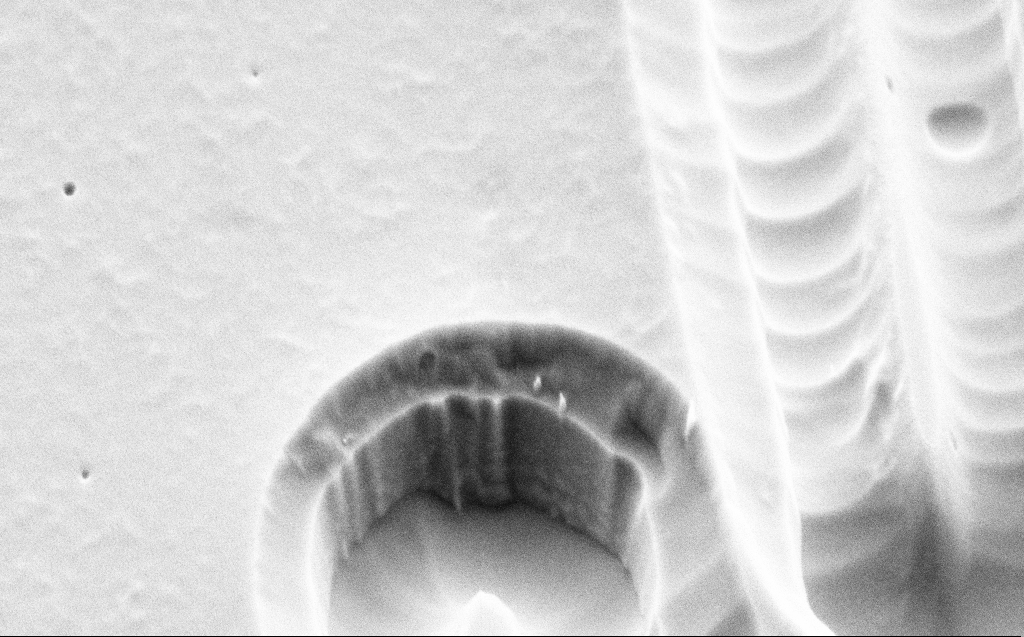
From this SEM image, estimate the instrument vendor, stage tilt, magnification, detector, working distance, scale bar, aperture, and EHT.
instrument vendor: Zeiss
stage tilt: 45°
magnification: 41.21 K X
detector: SE2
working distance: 6 mm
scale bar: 1000 nm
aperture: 30 µm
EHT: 3 kV